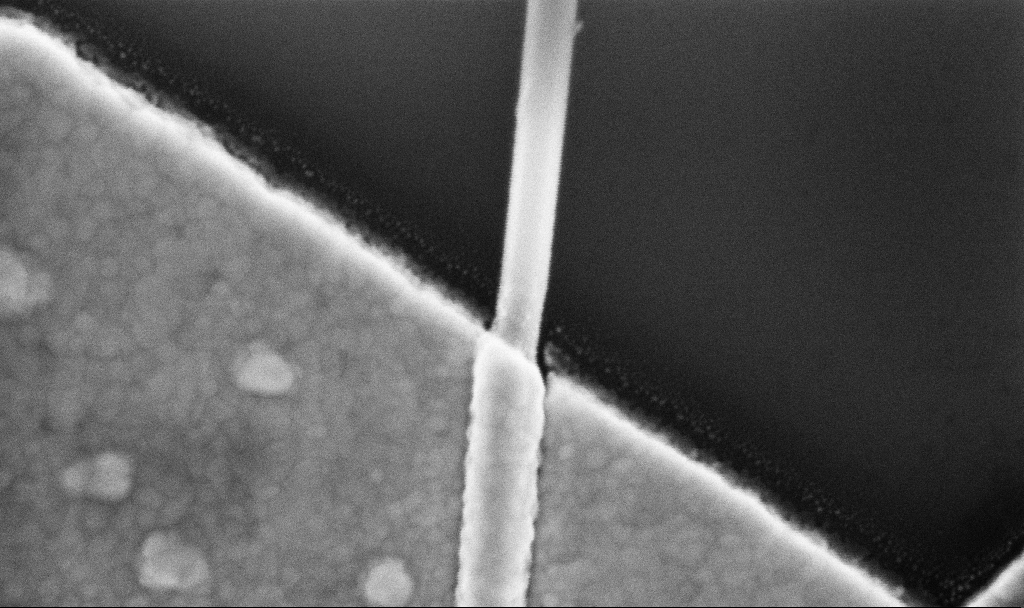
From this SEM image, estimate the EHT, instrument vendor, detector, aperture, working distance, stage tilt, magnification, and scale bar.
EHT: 5 kV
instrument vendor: Zeiss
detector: InLens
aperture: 30 µm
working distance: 8.7 mm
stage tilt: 0°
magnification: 200 K X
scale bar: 200 nm